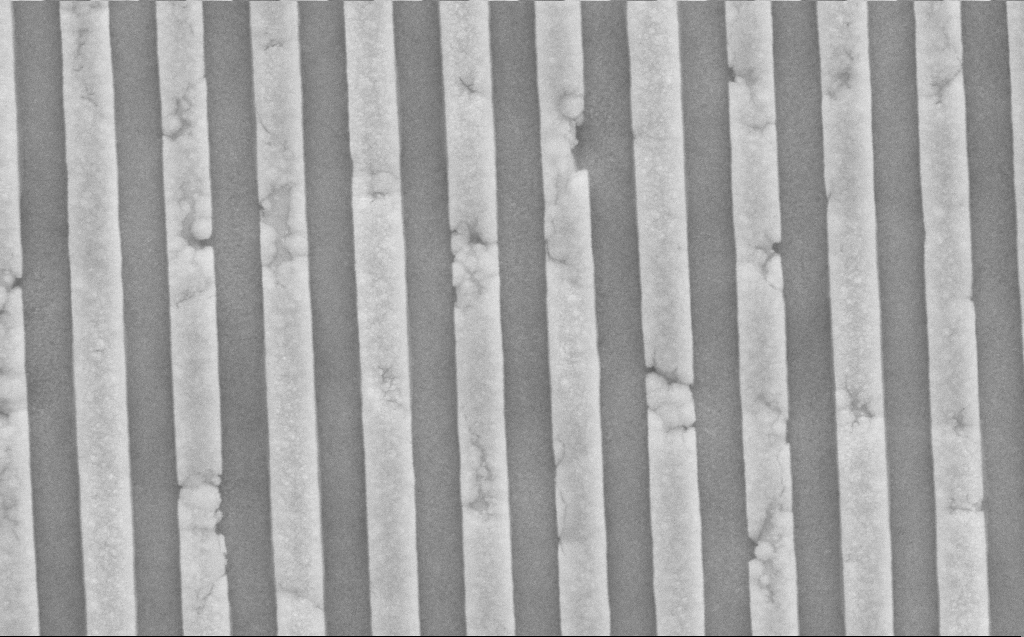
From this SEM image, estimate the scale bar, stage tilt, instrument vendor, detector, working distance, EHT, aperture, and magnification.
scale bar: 1000 nm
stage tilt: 0°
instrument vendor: Zeiss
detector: SE2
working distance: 10 mm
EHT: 10 kV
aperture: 30 µm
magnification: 70 K X